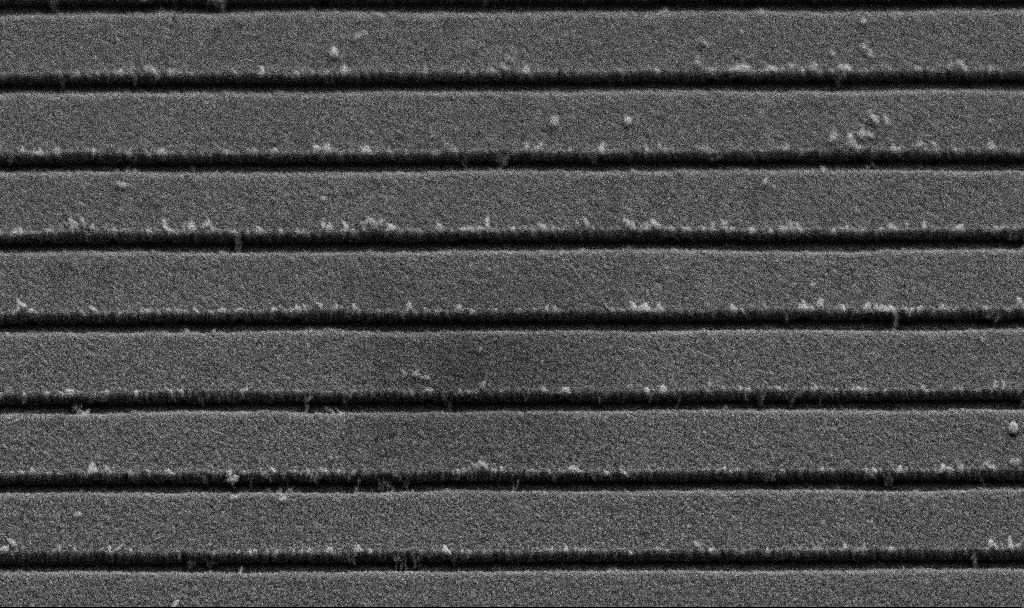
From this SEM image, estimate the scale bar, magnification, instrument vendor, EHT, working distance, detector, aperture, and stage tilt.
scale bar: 1000 nm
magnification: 51.28 K X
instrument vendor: Zeiss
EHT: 5 kV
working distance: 8.8 mm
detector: SE2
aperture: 30 µm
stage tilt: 45°